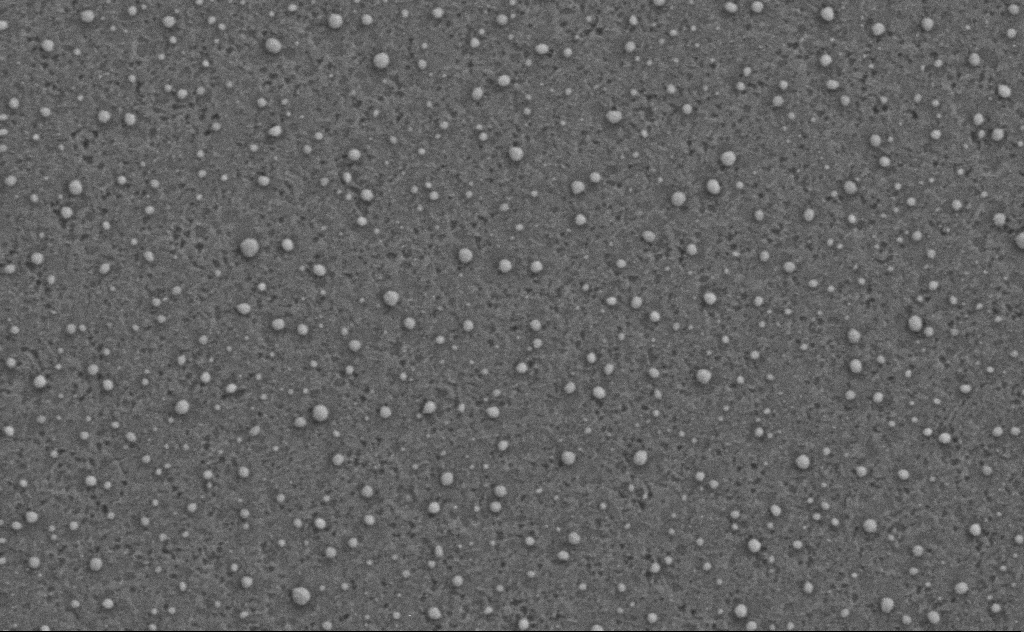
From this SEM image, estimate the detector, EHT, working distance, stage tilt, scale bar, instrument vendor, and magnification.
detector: SE2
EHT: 3 kV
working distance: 4 mm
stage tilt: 0°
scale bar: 200 nm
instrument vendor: Zeiss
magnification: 80 K X